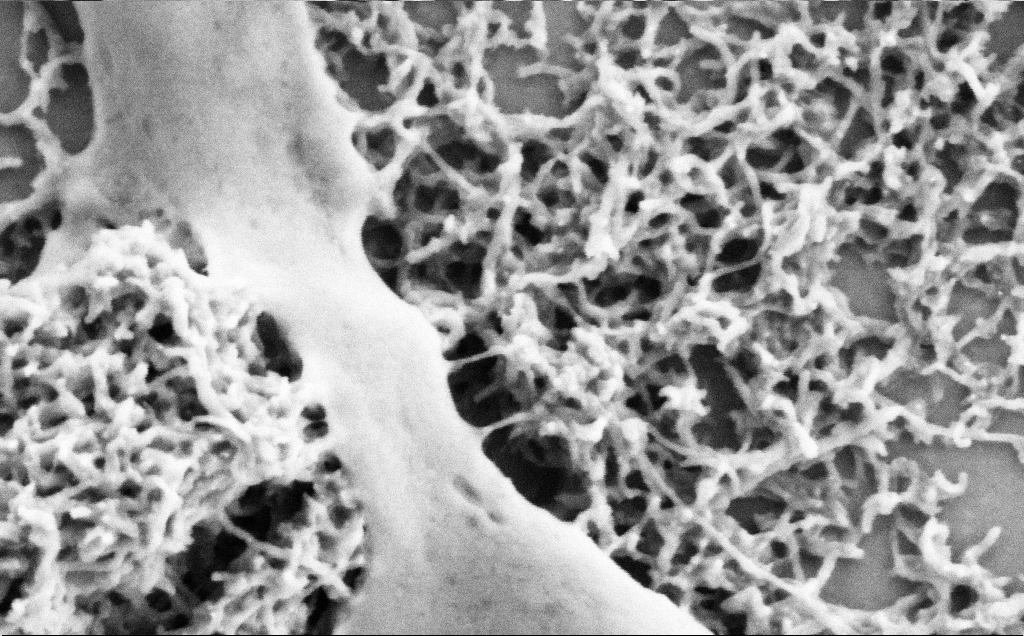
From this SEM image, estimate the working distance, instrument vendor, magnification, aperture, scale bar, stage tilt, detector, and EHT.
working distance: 7.1 mm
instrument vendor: Zeiss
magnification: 100 K X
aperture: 30 µm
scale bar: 200 nm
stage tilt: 0°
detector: SE2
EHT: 2 kV